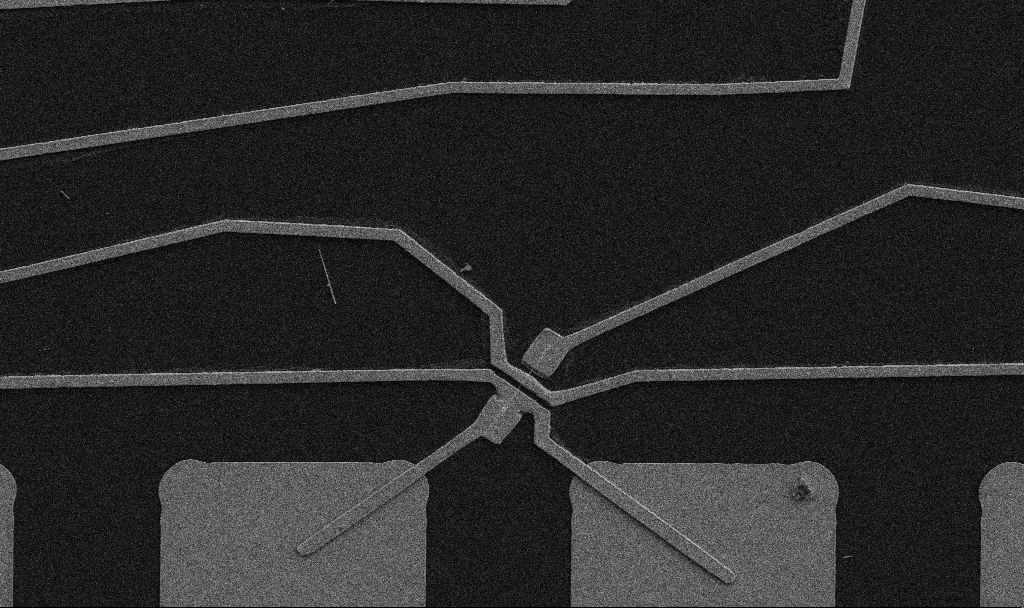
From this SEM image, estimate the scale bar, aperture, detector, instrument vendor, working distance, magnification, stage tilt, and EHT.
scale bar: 10000 nm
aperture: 30 µm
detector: SE2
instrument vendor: Zeiss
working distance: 10.7 mm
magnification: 5 K X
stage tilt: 0°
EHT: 5 kV